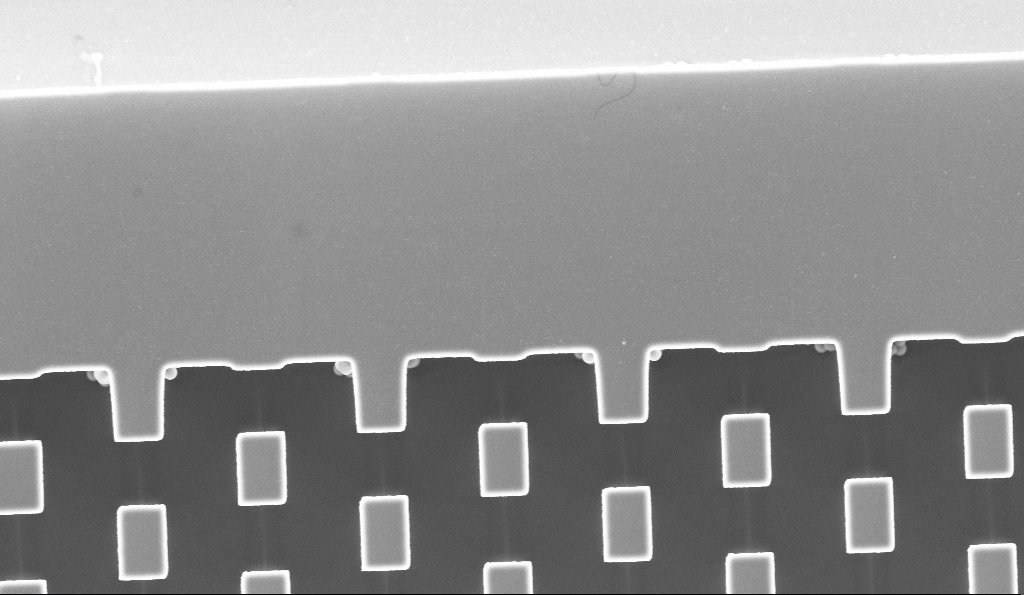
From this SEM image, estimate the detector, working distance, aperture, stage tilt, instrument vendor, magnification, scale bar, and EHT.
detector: InLens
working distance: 3.1 mm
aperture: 30 µm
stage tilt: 0°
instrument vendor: Zeiss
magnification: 8.57 K X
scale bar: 2000 nm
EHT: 5 kV